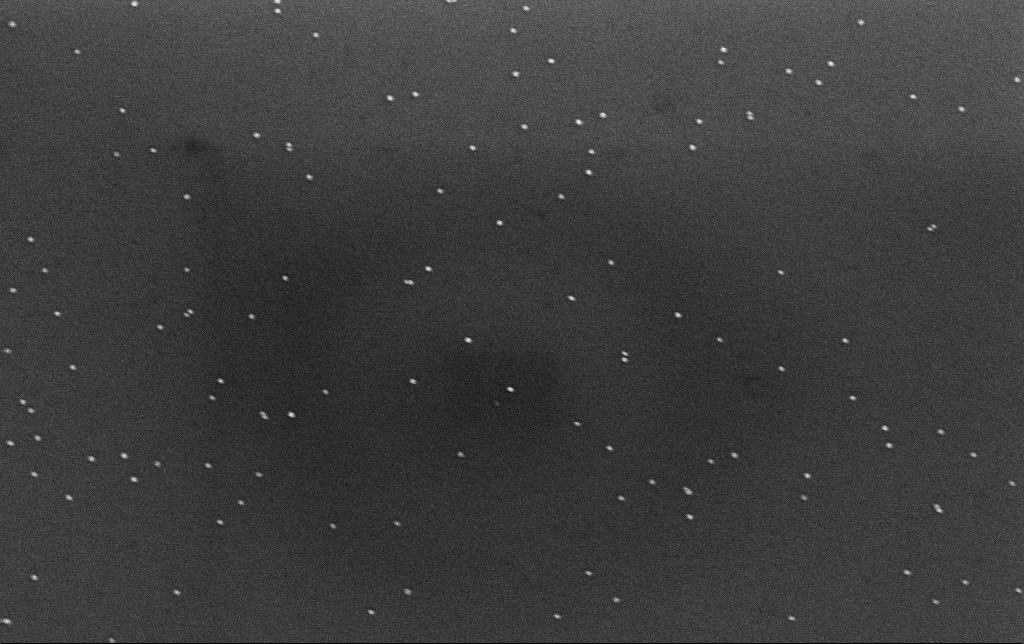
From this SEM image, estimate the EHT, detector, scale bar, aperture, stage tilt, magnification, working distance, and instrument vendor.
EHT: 10 kV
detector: InLens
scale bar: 200 nm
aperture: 30 µm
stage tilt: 0°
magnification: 100 K X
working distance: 3.1 mm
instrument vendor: Zeiss